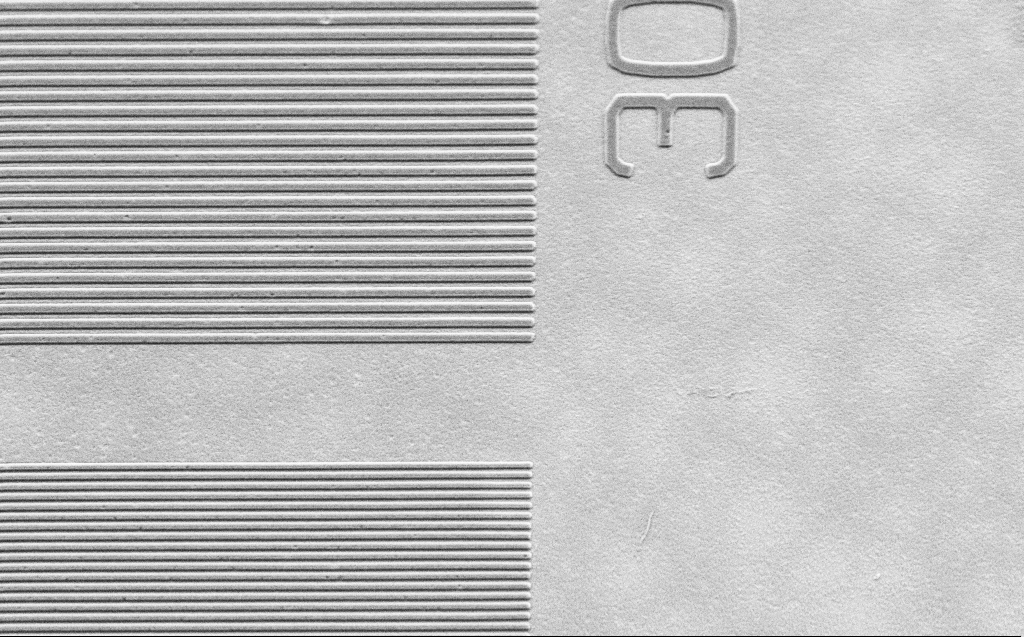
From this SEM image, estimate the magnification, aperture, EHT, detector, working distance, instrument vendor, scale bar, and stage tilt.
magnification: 9.5 K X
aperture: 30 µm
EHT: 2.5 kV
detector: SE2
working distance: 5 mm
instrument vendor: Zeiss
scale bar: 2000 nm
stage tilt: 30°